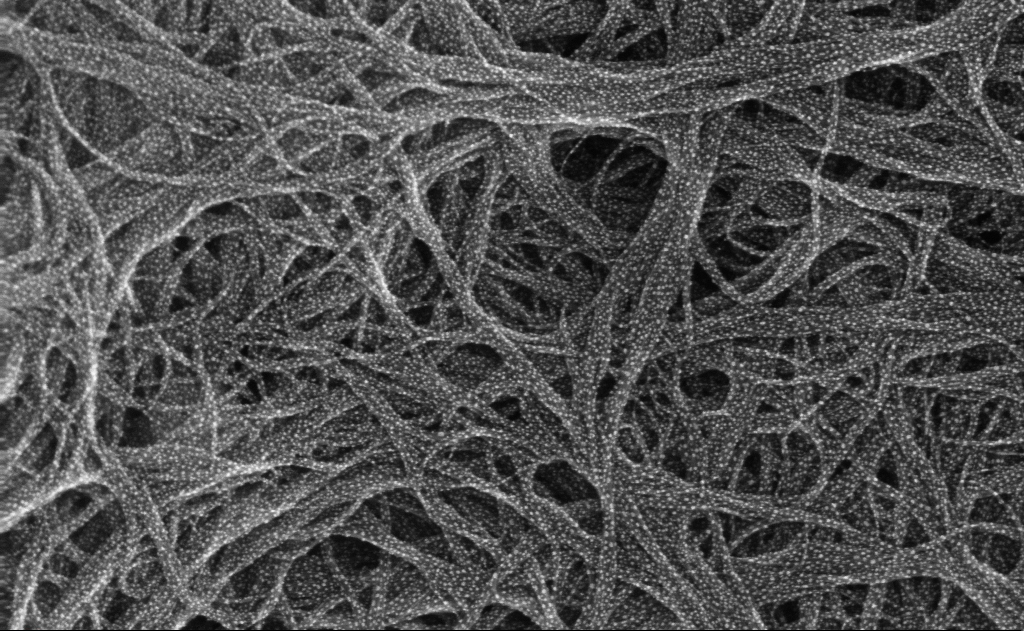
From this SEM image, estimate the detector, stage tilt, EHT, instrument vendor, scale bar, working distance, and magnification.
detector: InLens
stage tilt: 0°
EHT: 10 kV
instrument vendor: Zeiss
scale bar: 100 nm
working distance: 3 mm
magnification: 208.52 K X